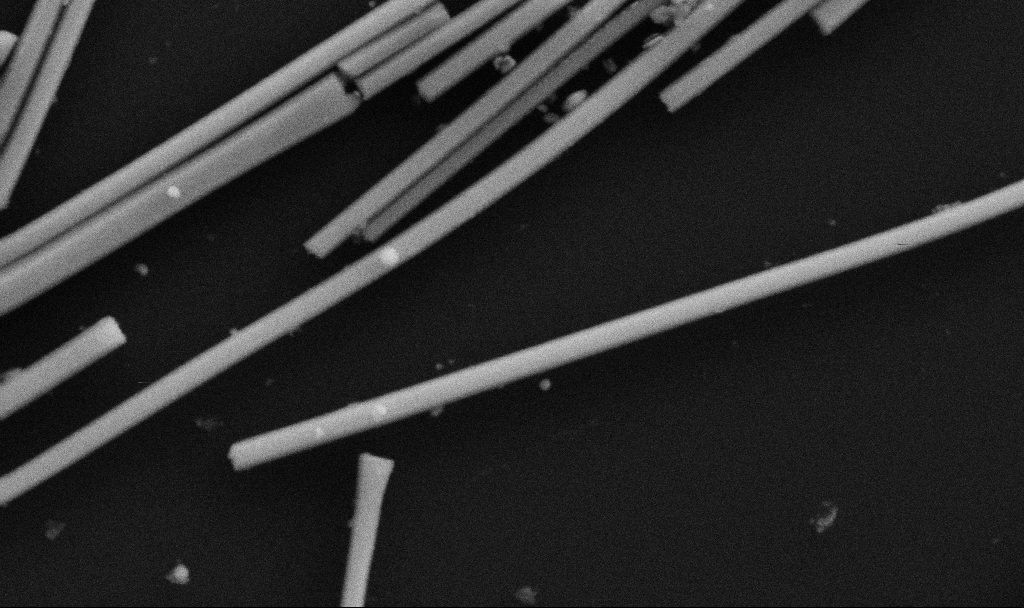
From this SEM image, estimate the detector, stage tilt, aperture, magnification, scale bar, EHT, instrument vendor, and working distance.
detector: SE2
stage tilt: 0°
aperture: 30 µm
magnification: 101.61 K X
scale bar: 200 nm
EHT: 5 kV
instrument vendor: Zeiss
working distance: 6.7 mm